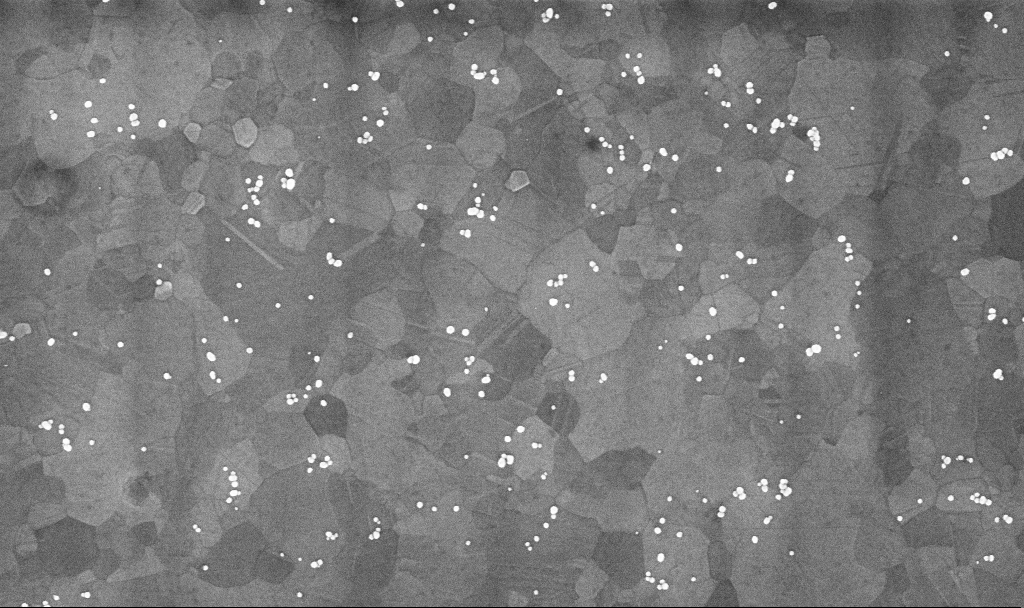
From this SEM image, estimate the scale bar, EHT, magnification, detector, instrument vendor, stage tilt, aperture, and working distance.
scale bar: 200 nm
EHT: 10 kV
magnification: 100.42 K X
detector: InLens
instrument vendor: Zeiss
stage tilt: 0°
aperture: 30 µm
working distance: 3.4 mm